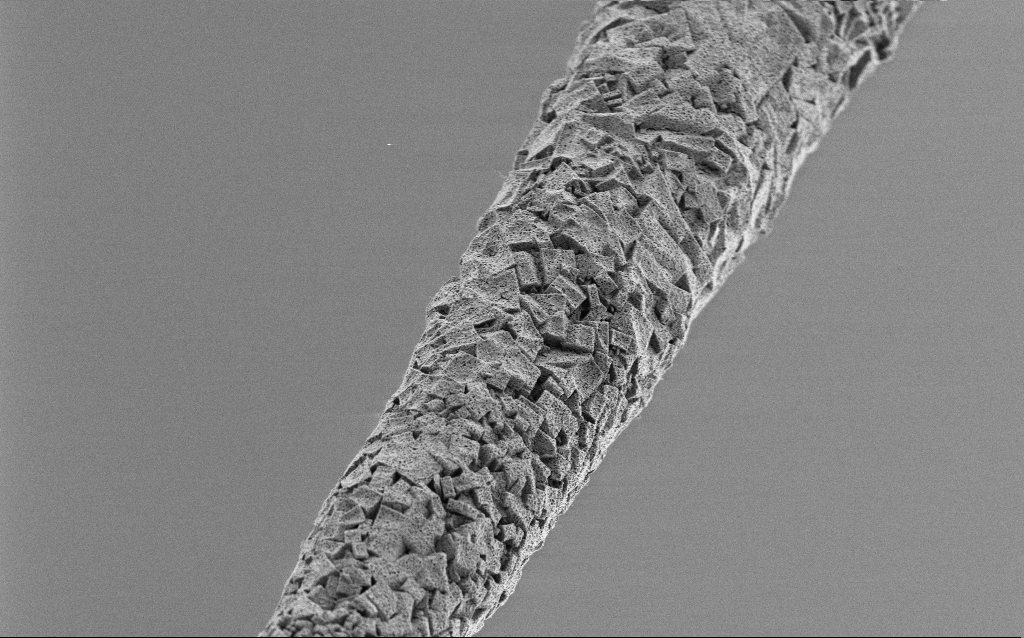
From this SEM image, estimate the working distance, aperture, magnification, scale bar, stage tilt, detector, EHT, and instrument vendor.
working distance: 6.8 mm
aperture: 30 µm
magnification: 5 K X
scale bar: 10000 nm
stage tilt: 45°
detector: SE2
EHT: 1 kV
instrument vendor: Zeiss